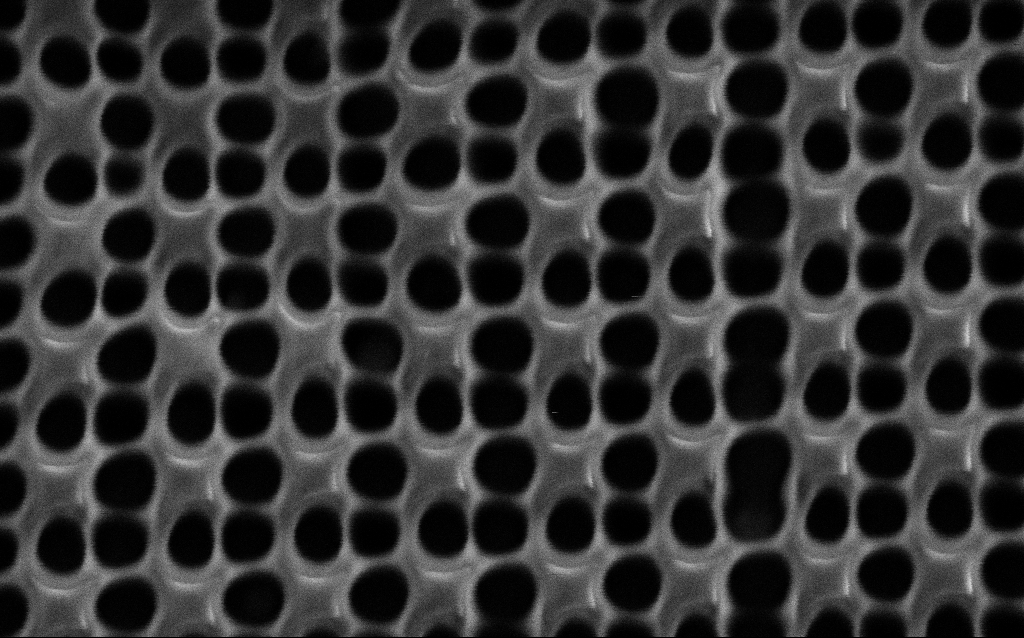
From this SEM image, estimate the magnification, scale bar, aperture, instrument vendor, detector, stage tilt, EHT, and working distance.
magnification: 99.75 K X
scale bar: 200 nm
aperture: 30 µm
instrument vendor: Zeiss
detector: SE2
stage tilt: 0°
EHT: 1.5 kV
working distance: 8 mm